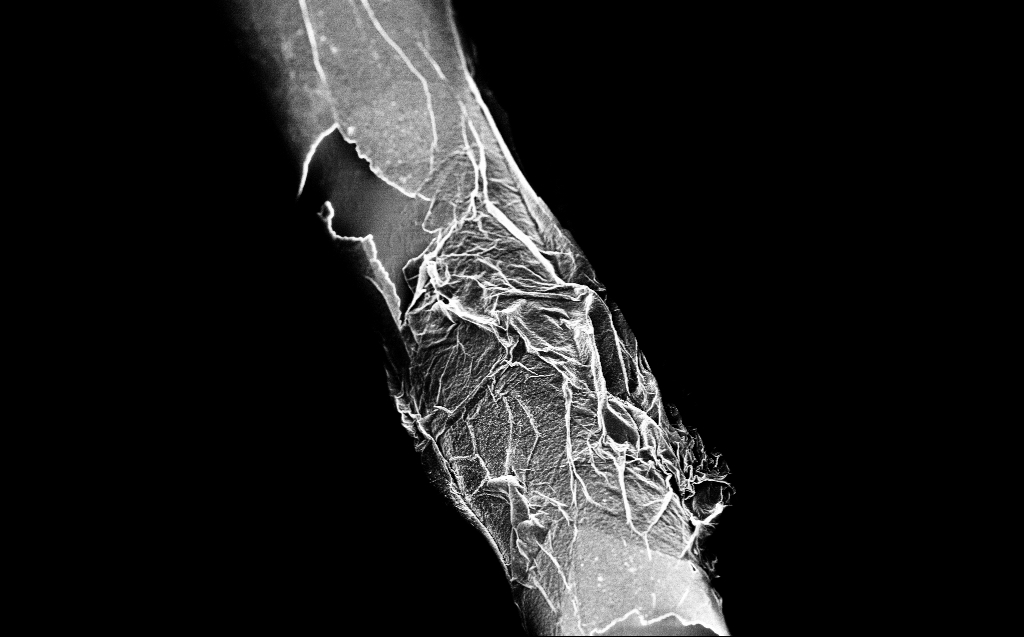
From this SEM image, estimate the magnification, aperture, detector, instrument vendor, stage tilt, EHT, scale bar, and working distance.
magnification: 5 K X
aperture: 30 µm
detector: InLens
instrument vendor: Zeiss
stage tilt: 45°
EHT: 2 kV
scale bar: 10000 nm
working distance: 3 mm